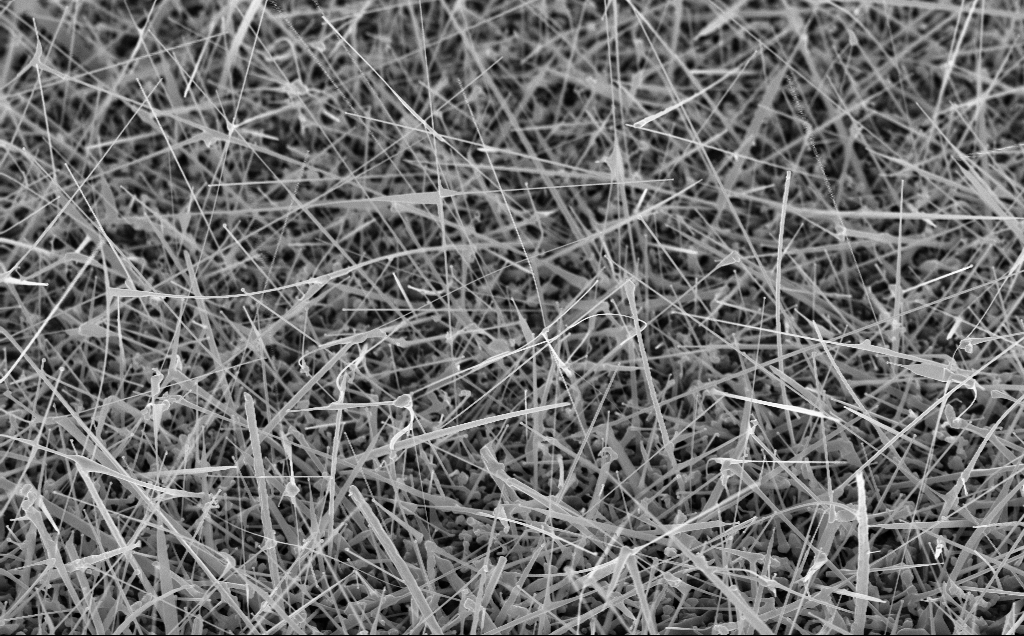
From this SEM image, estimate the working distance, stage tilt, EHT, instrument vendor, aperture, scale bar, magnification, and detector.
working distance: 8 mm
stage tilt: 45°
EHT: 10 kV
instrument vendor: Zeiss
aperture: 30 µm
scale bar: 2000 nm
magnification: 20 K X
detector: InLens